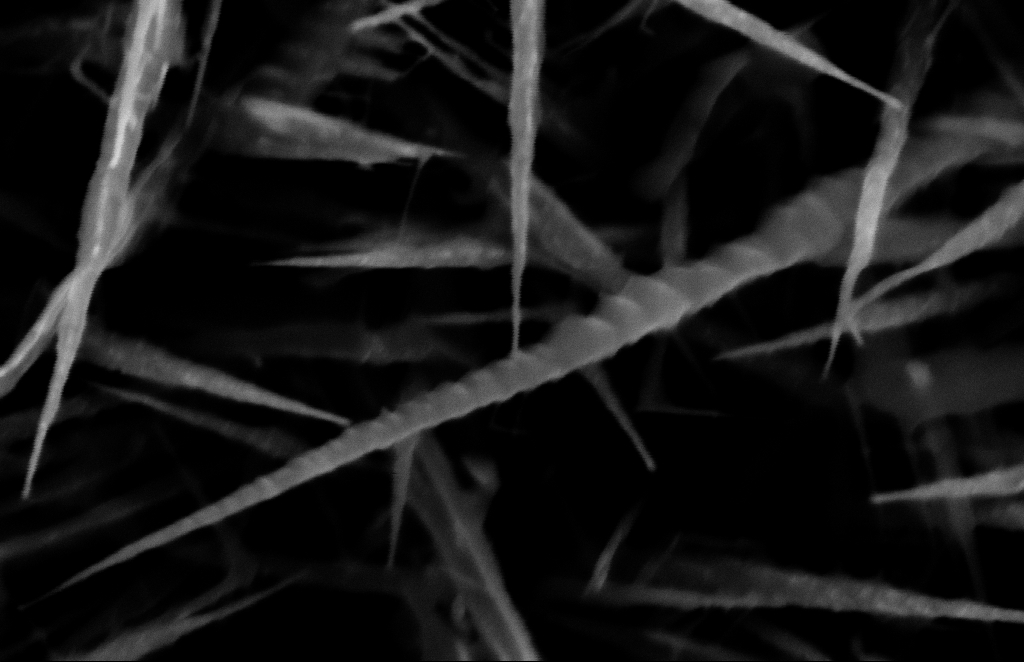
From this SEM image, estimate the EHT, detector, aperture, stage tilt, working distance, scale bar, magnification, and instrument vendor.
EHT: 10 kV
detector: InLens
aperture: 30 µm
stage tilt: -1.1°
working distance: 10 mm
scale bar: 100 nm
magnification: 246.75 K X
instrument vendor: Zeiss